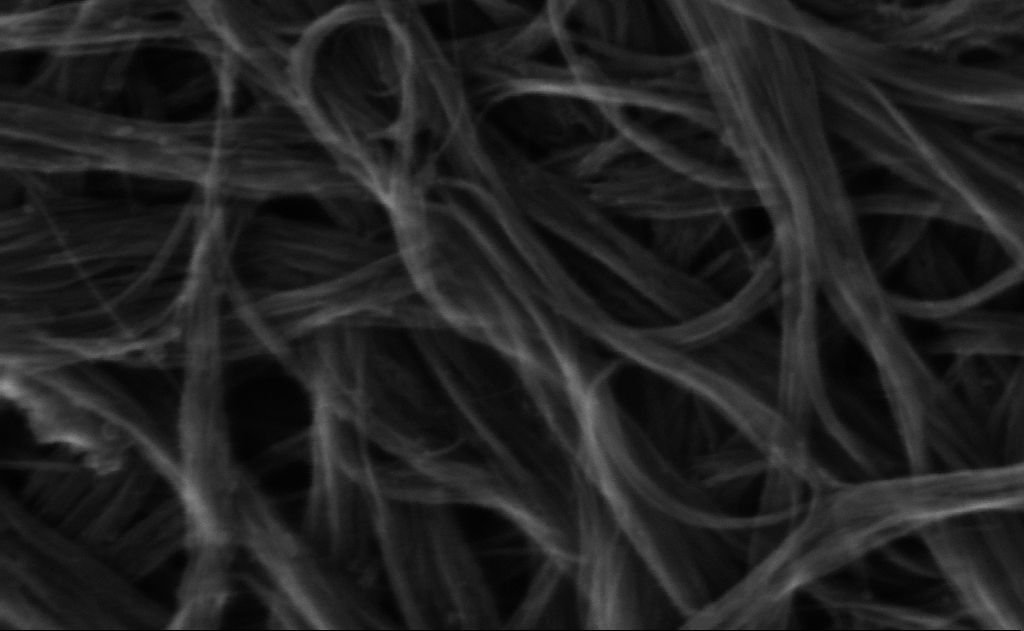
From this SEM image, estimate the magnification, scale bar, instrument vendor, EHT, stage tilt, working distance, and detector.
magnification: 596.32 K X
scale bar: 100 nm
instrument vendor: Zeiss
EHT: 10 kV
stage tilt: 0°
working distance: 3 mm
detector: InLens